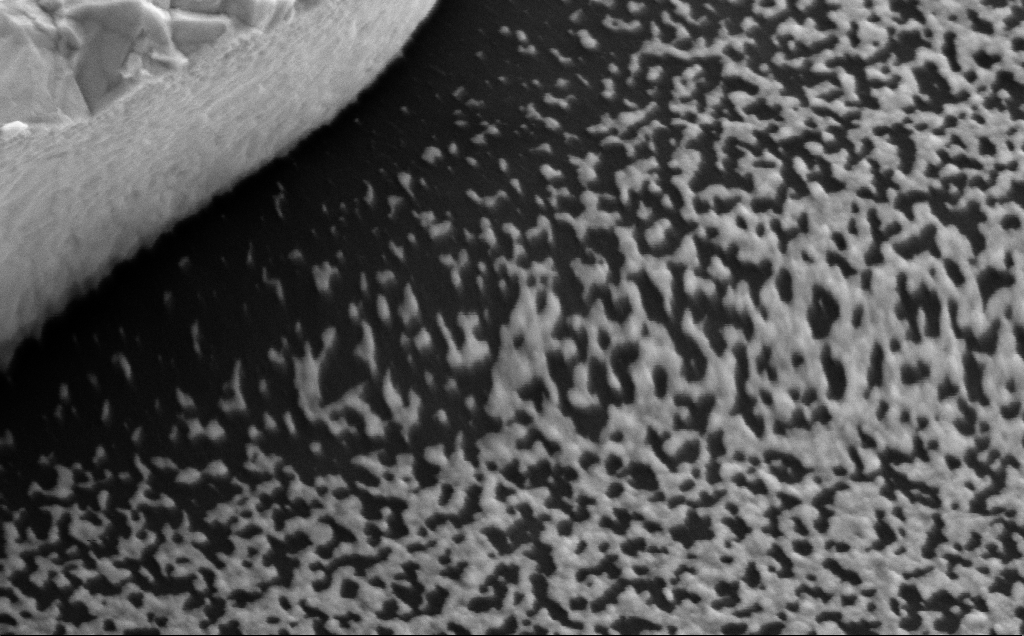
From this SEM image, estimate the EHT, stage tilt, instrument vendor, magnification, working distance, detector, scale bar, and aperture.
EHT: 5 kV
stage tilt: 35°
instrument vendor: Zeiss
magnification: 90.45 K X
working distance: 13 mm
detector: SE2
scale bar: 200 nm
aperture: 30 µm